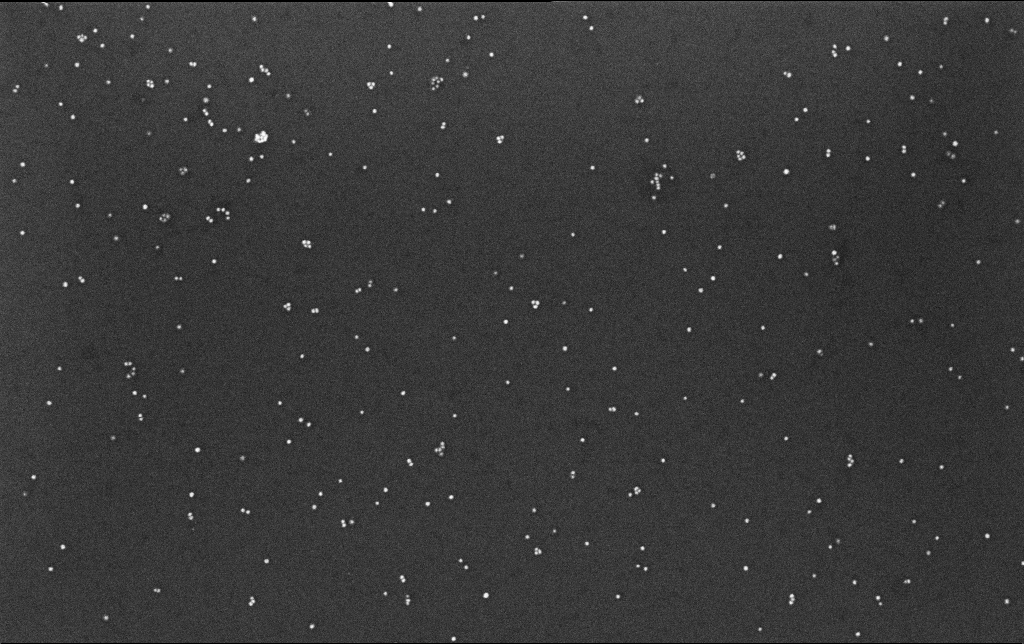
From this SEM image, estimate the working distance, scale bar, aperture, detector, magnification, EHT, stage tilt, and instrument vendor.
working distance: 3.4 mm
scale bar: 200 nm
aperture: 30 µm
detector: InLens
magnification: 100 K X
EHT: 10 kV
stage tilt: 0°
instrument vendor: Zeiss